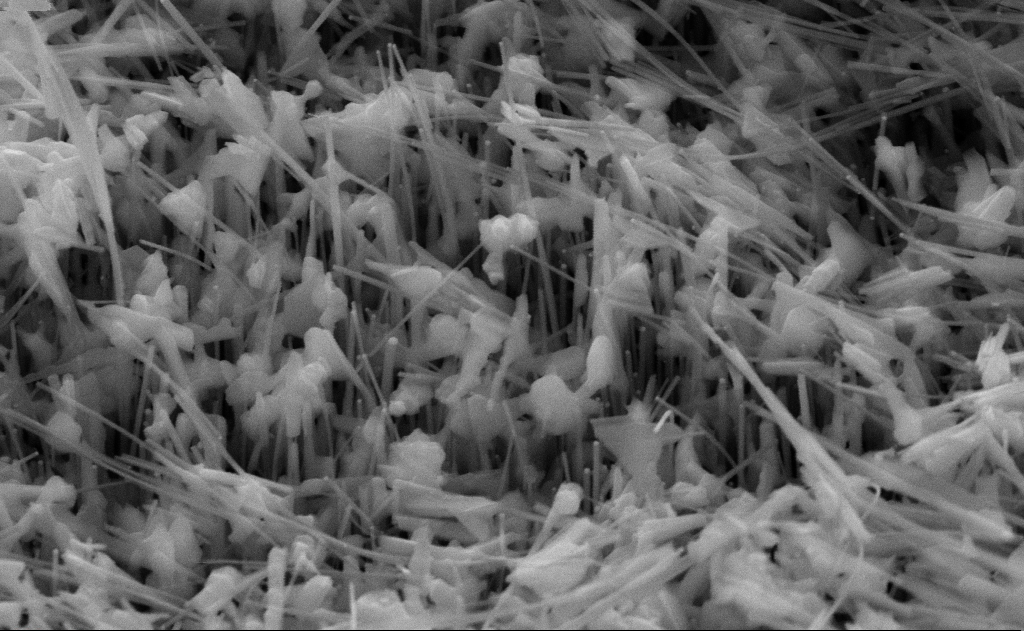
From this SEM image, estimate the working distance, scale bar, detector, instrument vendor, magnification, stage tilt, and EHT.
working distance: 11 mm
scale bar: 200 nm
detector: SE2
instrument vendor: Zeiss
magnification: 80 K X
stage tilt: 45°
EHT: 10 kV